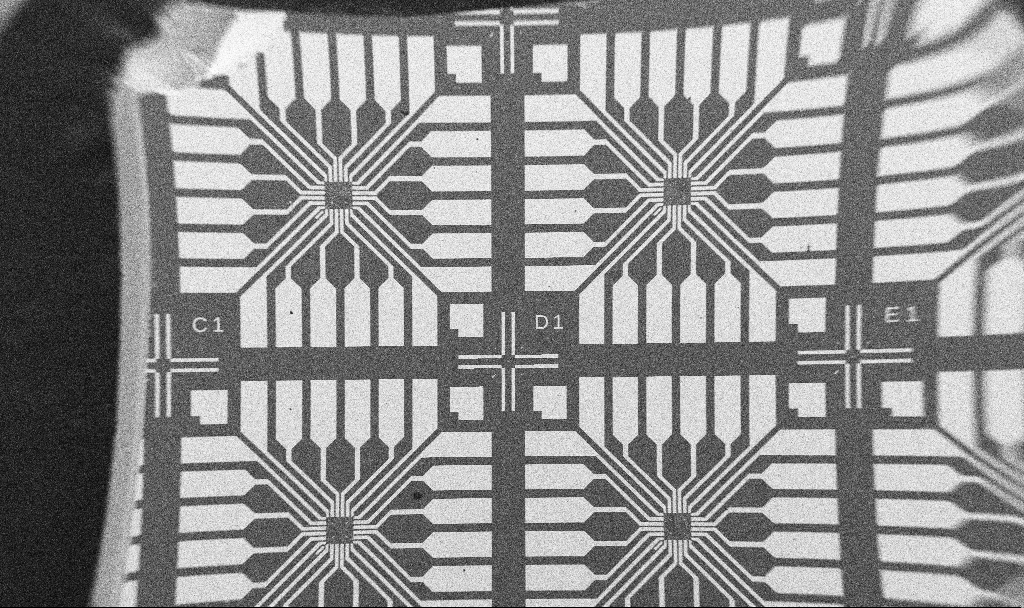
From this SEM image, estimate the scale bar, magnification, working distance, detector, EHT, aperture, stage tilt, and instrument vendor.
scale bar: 1e+06 nm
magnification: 0.061 K X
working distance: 10.7 mm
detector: SE2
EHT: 5 kV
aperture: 30 µm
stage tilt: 0°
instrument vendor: Zeiss